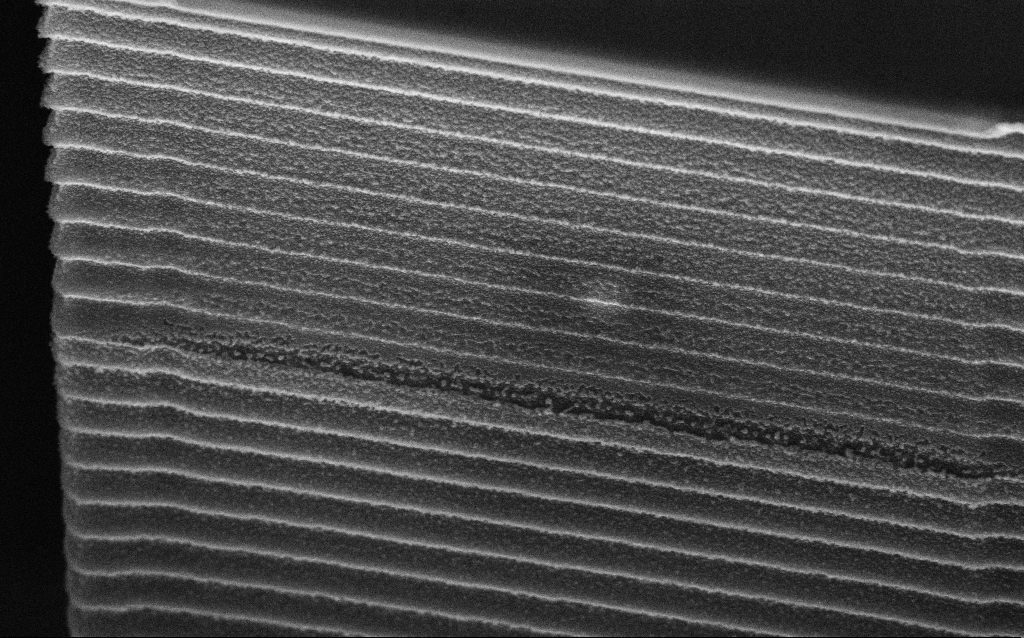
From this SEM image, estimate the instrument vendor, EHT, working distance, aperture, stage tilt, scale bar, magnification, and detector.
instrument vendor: Zeiss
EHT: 5 kV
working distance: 8 mm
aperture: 30 µm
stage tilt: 45°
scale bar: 1000 nm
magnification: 48.47 K X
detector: InLens